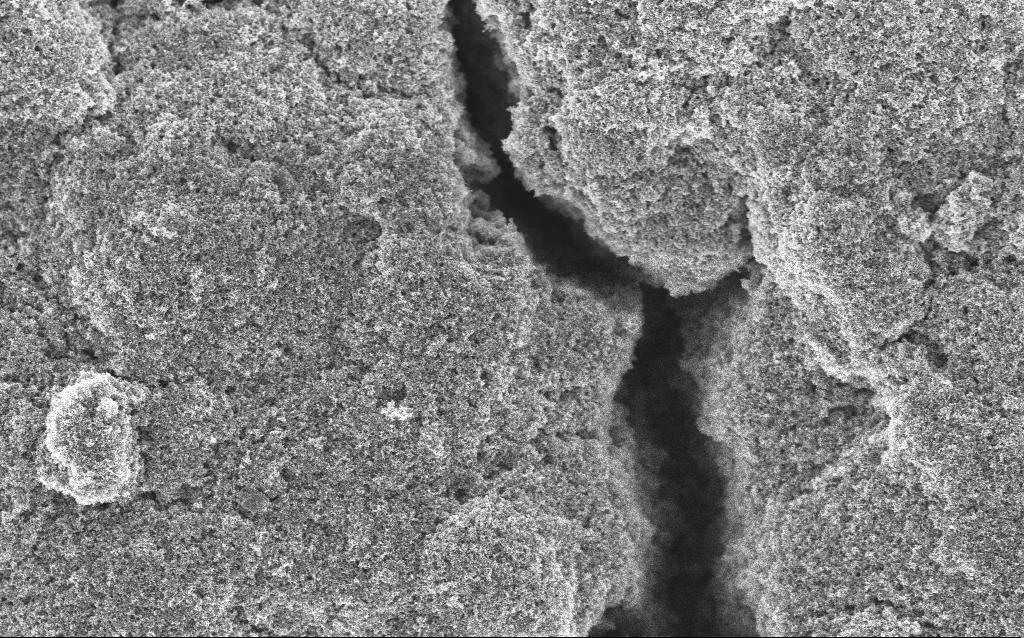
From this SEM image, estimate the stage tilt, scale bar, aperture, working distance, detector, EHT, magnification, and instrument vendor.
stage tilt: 0°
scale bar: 1000 nm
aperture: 30 µm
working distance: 4.2 mm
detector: InLens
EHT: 5 kV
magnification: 15.33 K X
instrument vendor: Zeiss